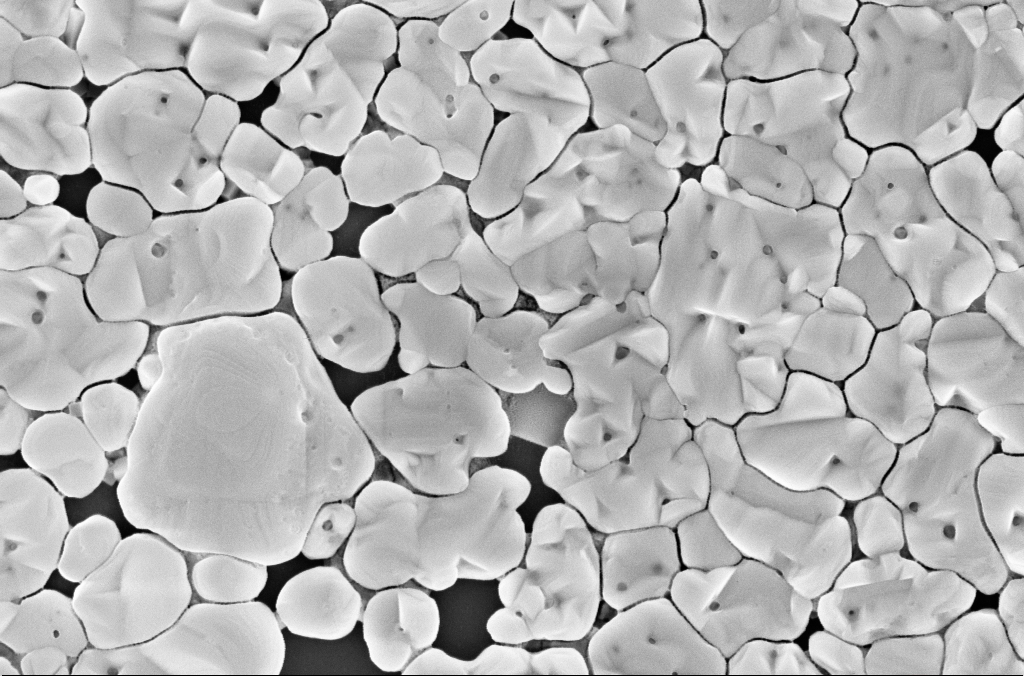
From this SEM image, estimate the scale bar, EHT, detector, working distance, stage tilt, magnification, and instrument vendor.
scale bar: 1000 nm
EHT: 5 kV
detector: InLens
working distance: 3 mm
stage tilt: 0°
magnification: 50 K X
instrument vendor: Zeiss